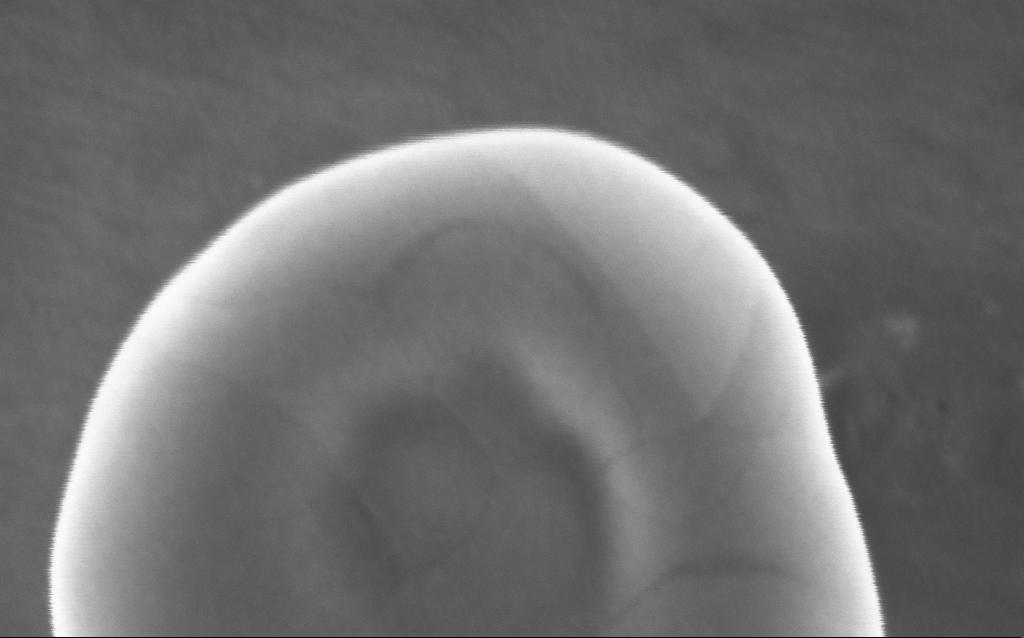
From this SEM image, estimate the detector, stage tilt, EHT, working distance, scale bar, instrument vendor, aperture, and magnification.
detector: InLens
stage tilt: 0°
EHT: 5 kV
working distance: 2 mm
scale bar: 100 nm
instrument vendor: Zeiss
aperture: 30 µm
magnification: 451 K X